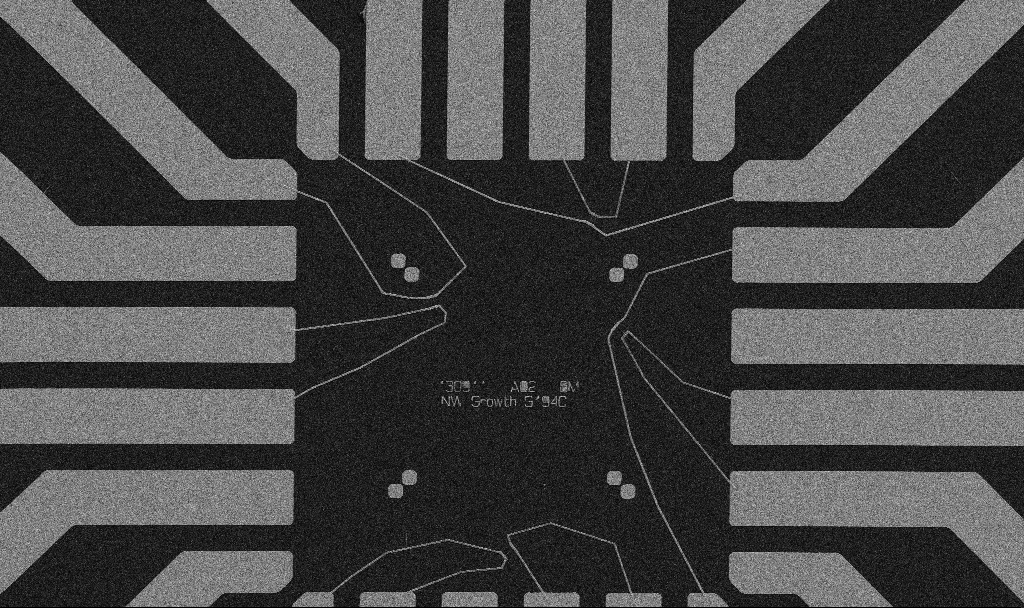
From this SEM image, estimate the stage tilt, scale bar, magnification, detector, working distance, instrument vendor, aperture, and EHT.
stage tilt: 0°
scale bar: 20000 nm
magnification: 1 K X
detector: SE2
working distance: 10.7 mm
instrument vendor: Zeiss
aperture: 30 µm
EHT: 5 kV